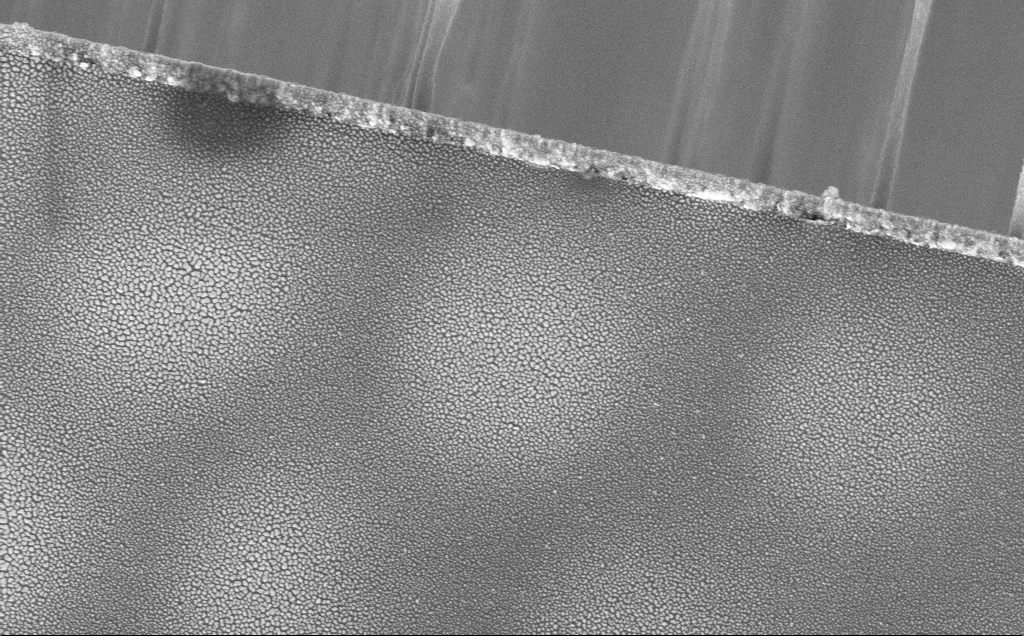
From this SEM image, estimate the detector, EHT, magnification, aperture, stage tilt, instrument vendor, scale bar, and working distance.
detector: InLens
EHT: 5 kV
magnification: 40.99 K X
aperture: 30 µm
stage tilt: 0°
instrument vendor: Zeiss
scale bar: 1000 nm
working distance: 11 mm